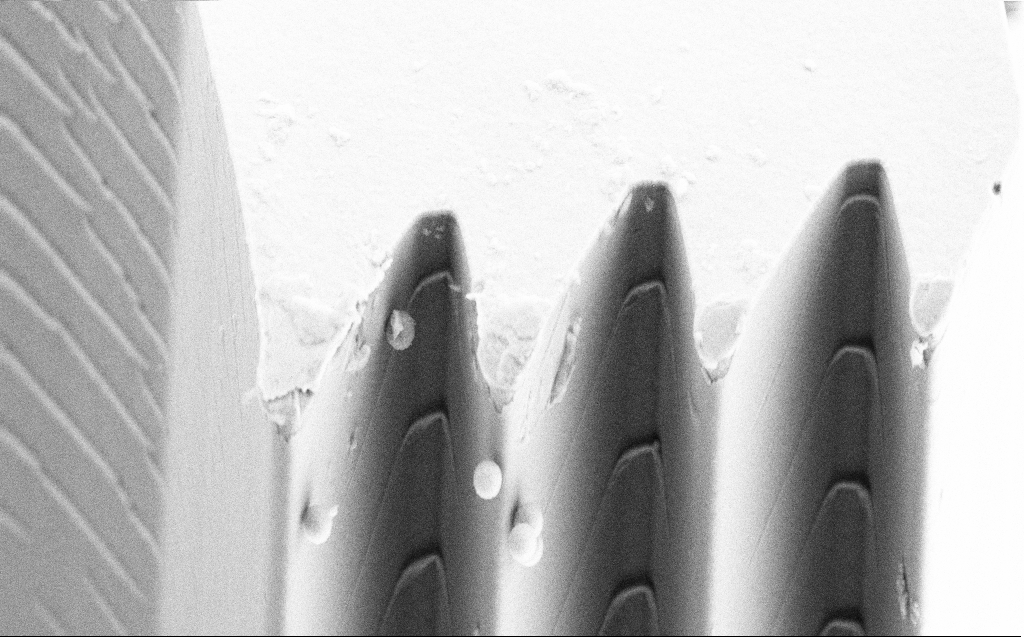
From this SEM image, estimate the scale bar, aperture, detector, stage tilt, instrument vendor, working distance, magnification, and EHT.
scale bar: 10000 nm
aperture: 30 µm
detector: SE2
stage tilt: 45°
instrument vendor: Zeiss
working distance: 5 mm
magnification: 7.12 K X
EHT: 10 kV